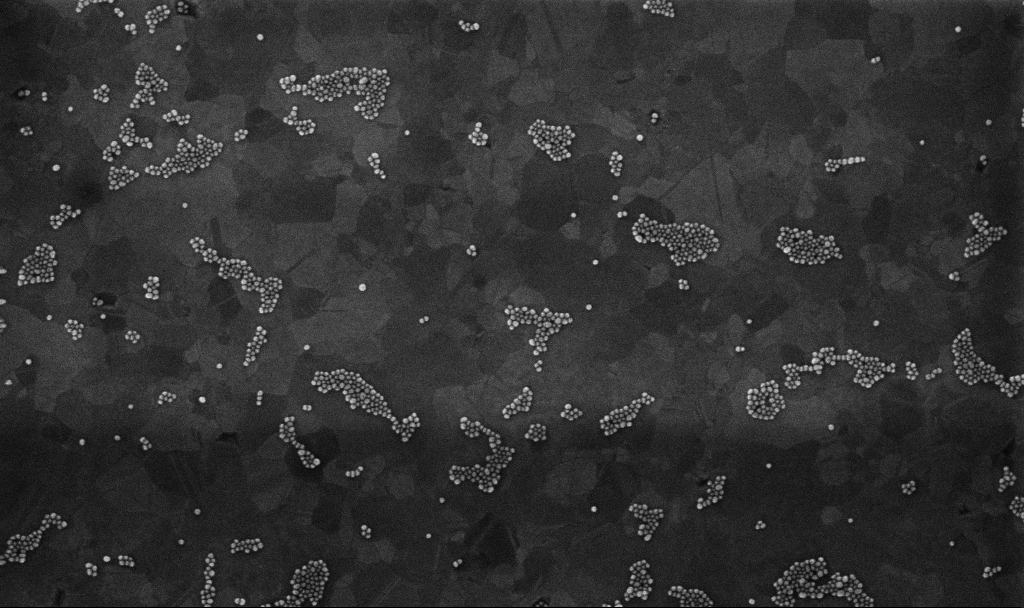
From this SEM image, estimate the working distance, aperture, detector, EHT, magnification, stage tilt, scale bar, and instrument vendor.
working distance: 3.2 mm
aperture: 30 µm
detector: InLens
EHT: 10 kV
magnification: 100 K X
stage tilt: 0°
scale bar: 200 nm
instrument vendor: Zeiss